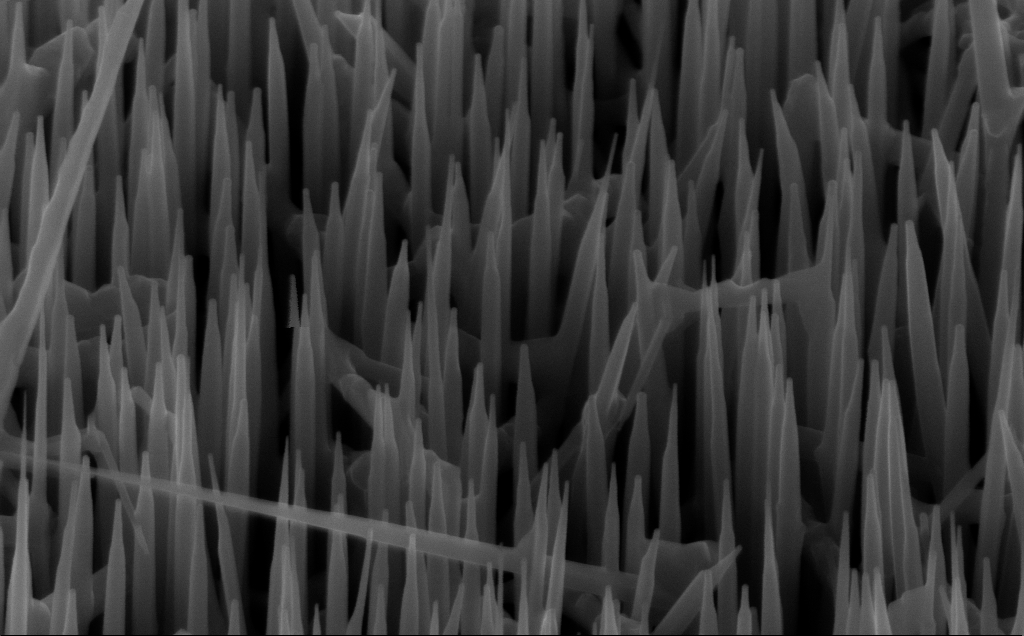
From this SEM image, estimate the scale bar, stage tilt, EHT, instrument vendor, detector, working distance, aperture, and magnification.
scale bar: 200 nm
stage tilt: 45°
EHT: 10 kV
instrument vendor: Zeiss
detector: InLens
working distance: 6 mm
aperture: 30 µm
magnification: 80 K X